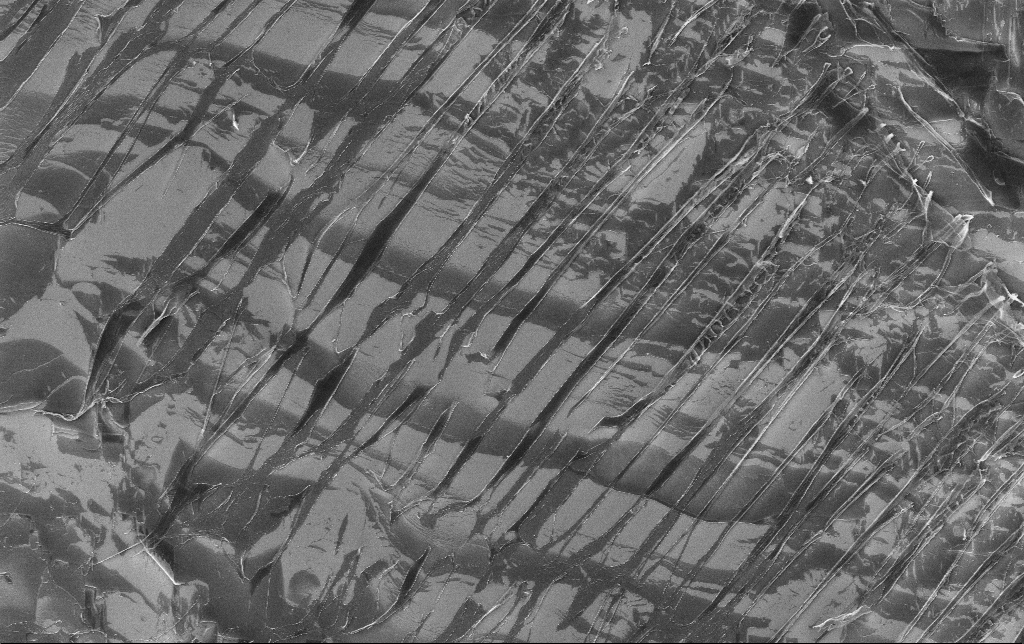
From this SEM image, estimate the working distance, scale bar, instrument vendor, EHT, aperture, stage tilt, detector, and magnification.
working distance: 3.1 mm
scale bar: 10000 nm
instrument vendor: Zeiss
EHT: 10 kV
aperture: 30 µm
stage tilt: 0°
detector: InLens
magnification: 2.18 K X